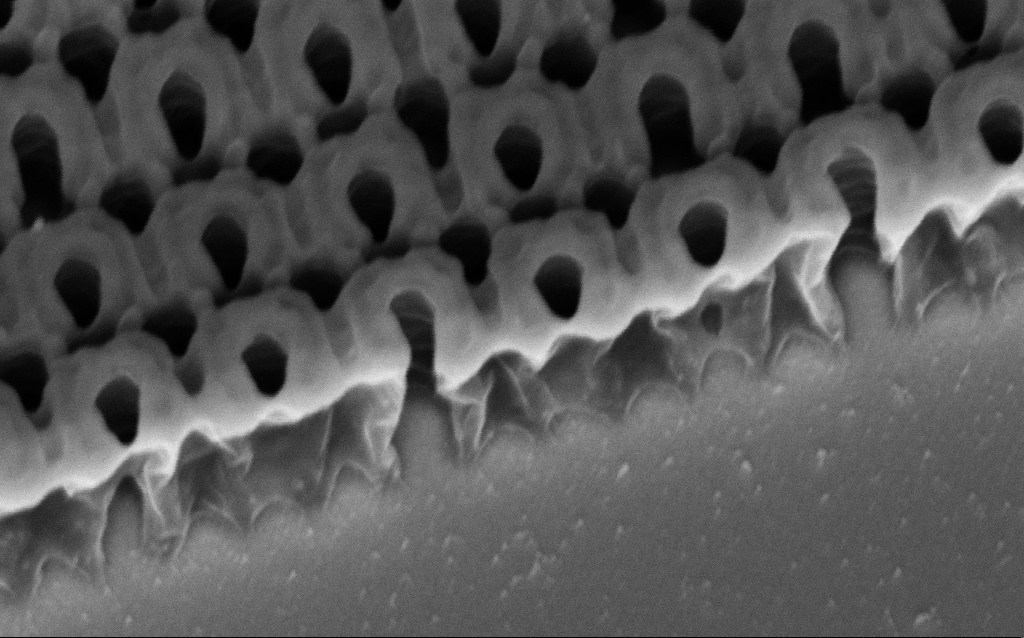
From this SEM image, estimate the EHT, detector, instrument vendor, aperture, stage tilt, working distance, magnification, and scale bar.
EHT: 3 kV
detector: InLens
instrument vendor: Zeiss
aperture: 30 µm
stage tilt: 45°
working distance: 7.3 mm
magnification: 122.81 K X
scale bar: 200 nm